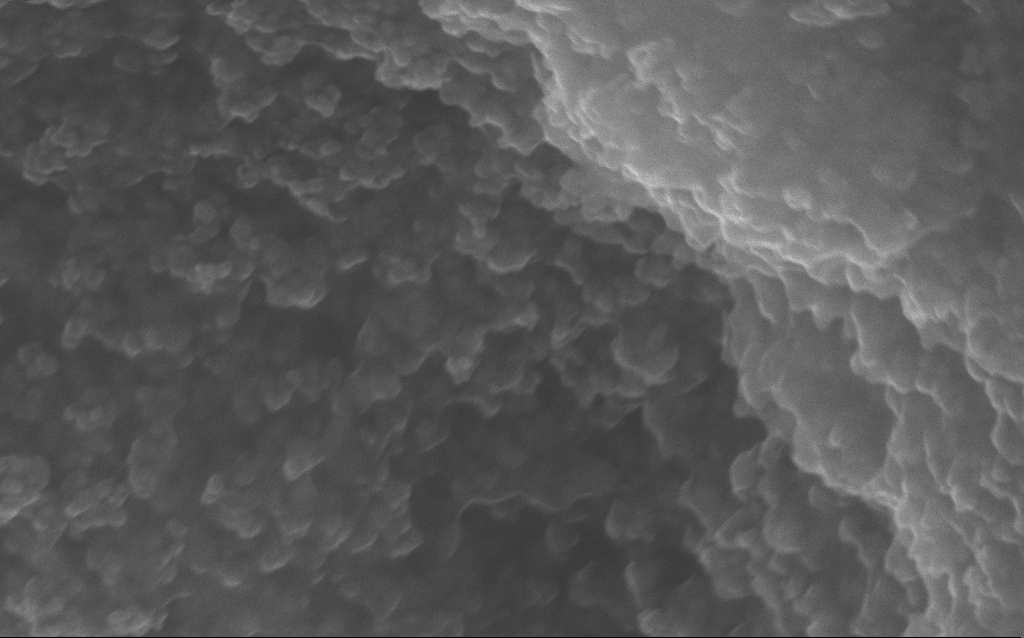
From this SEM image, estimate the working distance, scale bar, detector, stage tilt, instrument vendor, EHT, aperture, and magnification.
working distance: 2.8 mm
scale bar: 100 nm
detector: InLens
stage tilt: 0°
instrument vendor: Zeiss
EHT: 10 kV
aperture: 30 µm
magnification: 204.13 K X